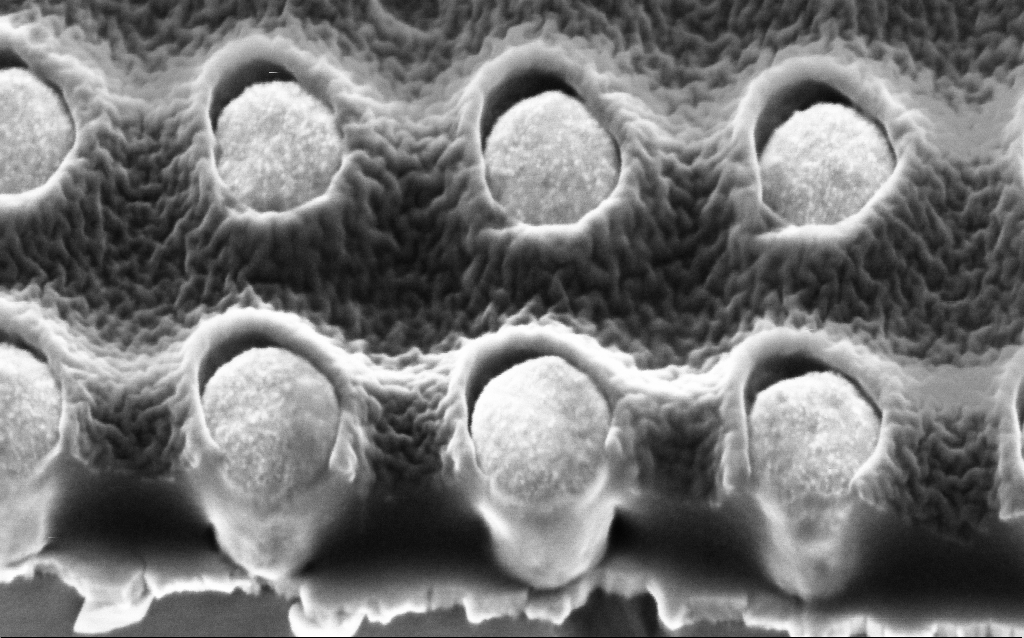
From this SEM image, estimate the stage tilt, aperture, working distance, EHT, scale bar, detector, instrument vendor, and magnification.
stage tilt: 45°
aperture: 30 µm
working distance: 3.5 mm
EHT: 2 kV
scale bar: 200 nm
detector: InLens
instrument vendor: Zeiss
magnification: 202.05 K X